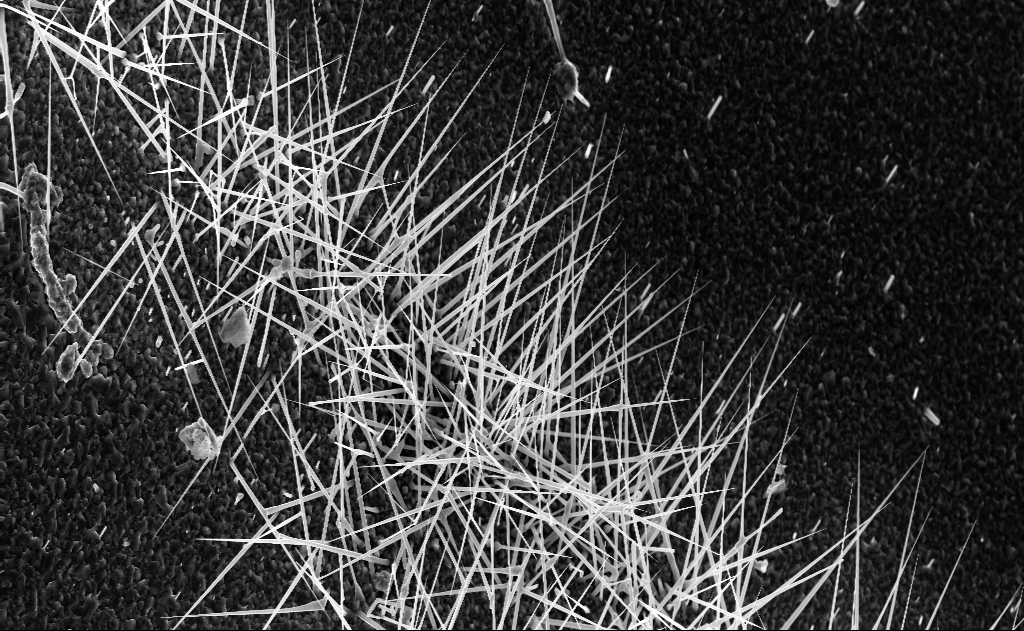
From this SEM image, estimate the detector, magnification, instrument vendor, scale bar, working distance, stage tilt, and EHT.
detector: InLens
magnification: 5 K X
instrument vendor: Zeiss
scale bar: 10000 nm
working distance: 4 mm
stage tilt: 0°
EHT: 10 kV